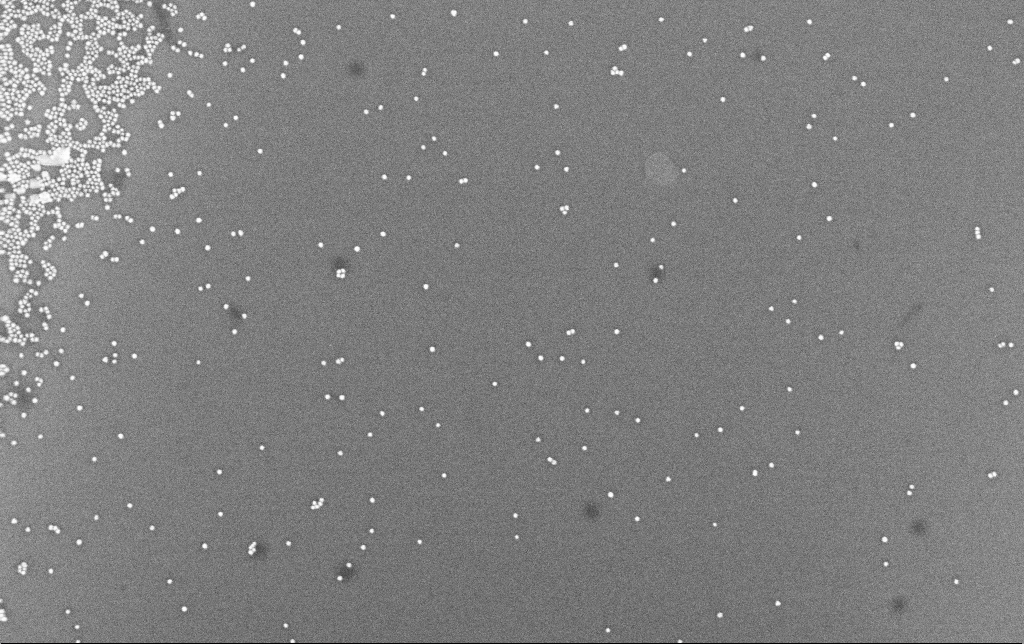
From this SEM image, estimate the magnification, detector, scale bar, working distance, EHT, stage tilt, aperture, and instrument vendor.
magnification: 100 K X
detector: InLens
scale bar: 200 nm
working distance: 6.6 mm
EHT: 8 kV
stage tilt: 0°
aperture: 30 µm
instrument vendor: Zeiss